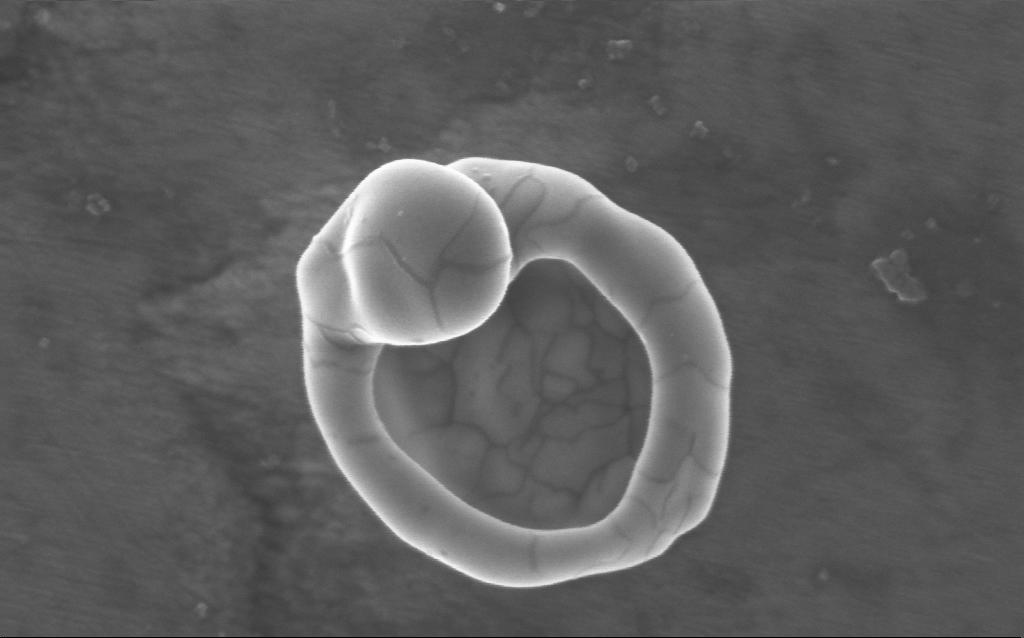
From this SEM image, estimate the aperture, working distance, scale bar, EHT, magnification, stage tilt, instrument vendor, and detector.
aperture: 30 µm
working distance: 2 mm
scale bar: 100 nm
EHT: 5 kV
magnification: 170 K X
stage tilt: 0°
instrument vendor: Zeiss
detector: InLens